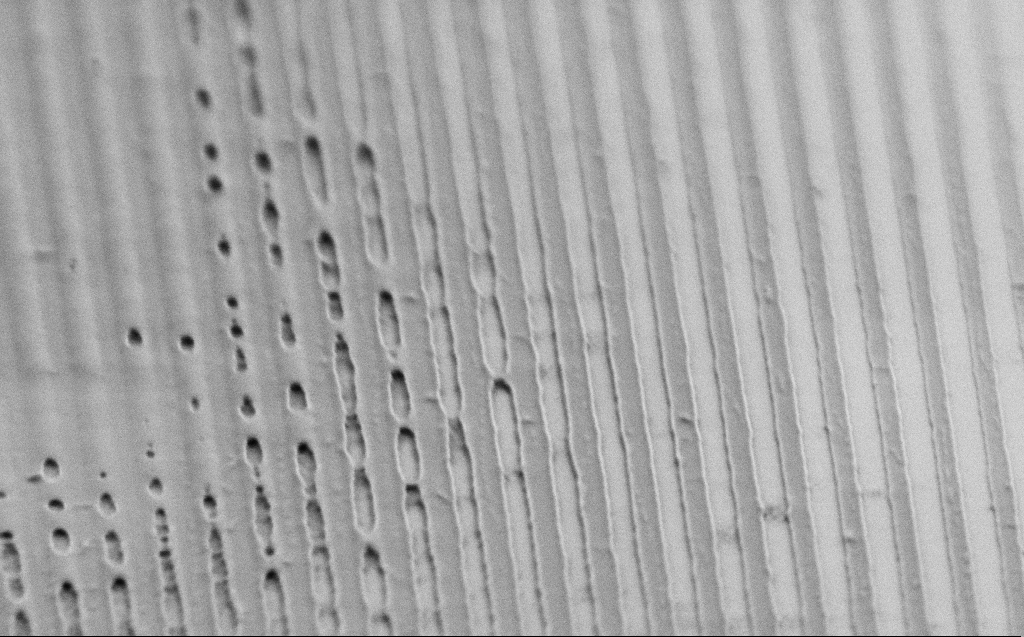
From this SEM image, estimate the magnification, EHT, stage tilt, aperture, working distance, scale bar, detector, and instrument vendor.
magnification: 38.15 K X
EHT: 1 kV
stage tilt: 60.7°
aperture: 30 µm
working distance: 3 mm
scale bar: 1000 nm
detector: SE2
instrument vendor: Zeiss